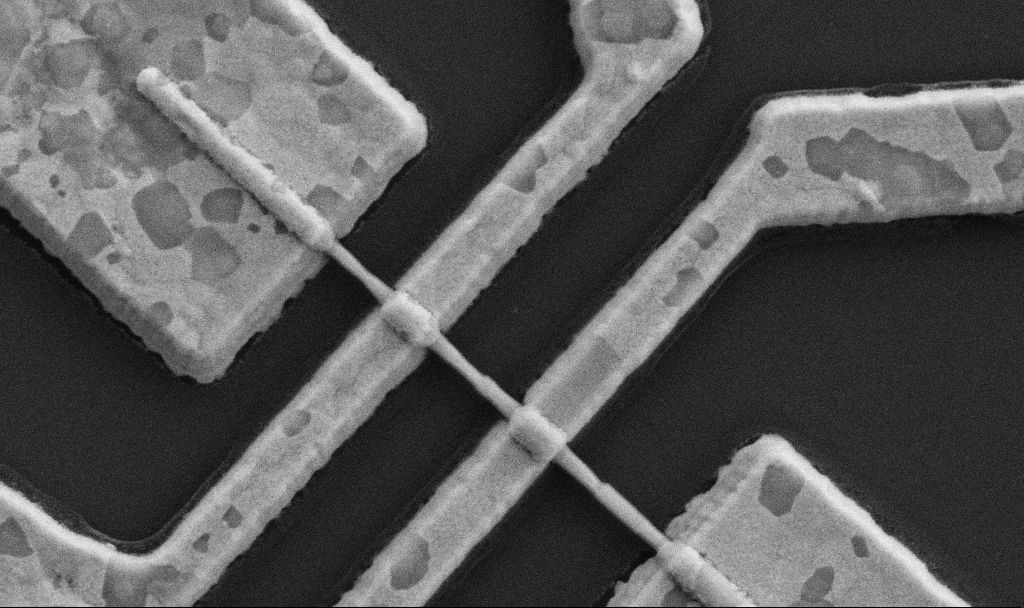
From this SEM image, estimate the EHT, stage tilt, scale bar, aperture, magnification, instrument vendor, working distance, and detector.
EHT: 5 kV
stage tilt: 0°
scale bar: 1000 nm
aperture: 30 µm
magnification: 60 K X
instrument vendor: Zeiss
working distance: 10.7 mm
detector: SE2